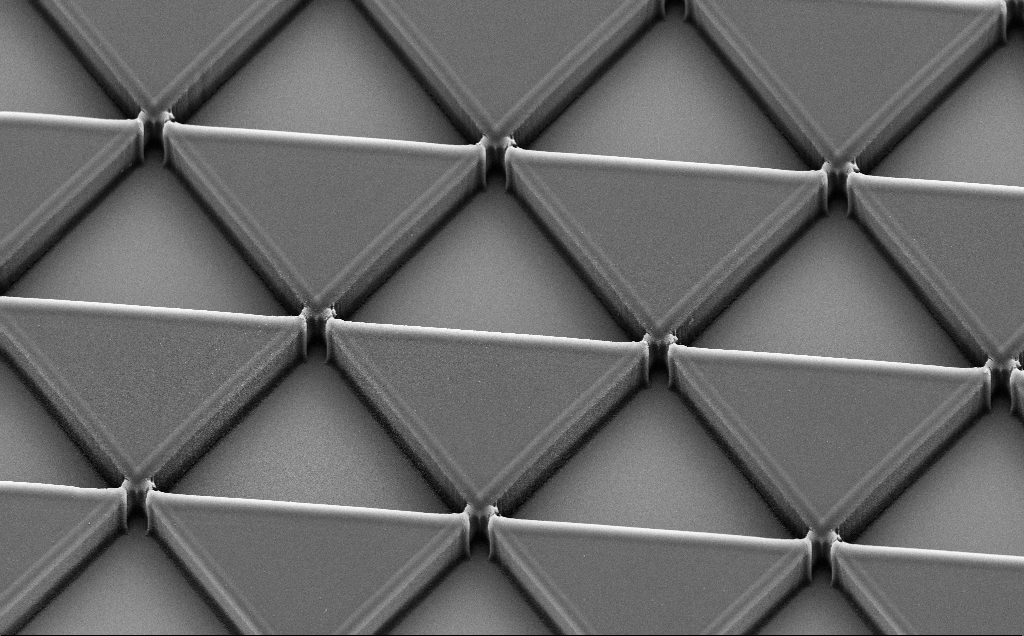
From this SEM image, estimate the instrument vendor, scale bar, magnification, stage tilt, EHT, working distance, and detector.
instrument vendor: Zeiss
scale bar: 10000 nm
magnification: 1.34 K X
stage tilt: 35.3°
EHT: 10 kV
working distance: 11 mm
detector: SE2